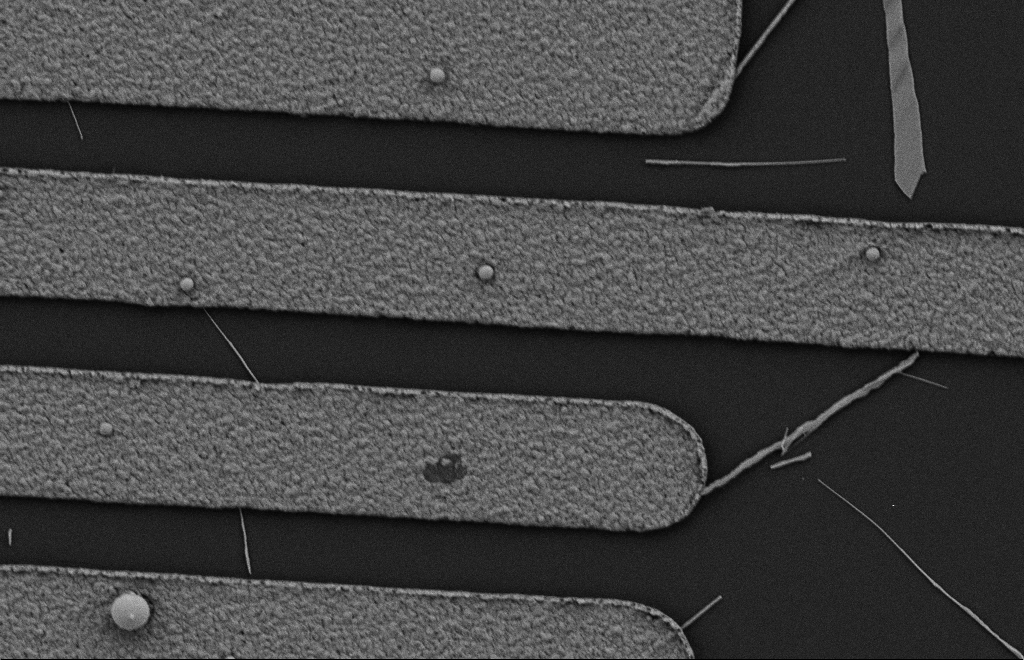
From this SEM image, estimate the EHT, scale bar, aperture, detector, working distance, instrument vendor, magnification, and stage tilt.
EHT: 2 kV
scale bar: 2000 nm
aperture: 20 µm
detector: SE2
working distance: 10 mm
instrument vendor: Zeiss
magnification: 18.28 K X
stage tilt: -0.3°